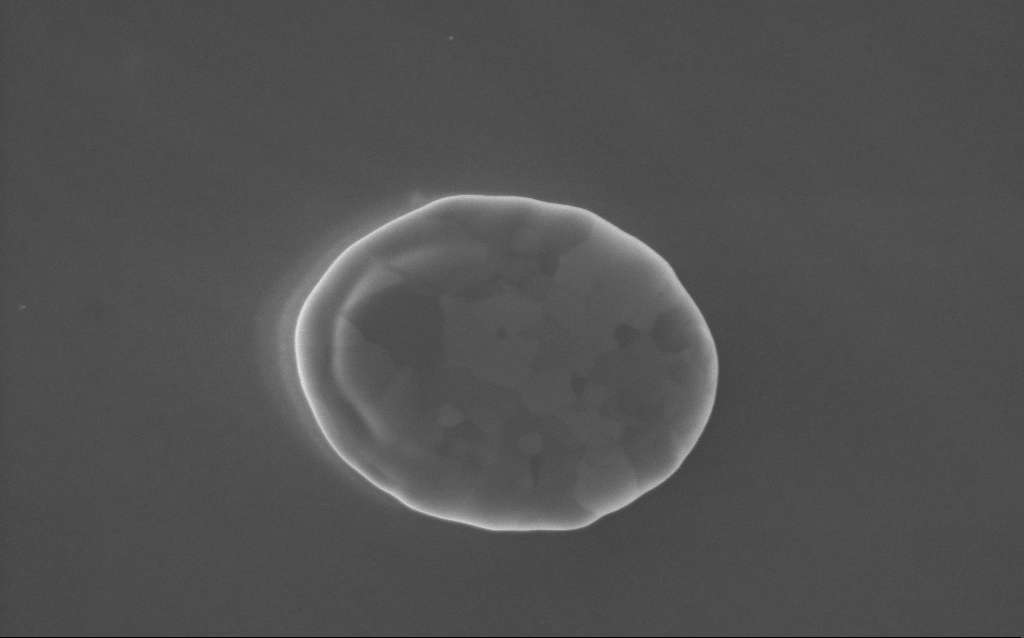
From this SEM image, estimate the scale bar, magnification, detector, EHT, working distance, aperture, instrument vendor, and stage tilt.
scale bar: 1000 nm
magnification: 52.76 K X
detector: InLens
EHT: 5 kV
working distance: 3 mm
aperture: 30 µm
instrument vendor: Zeiss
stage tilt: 0°